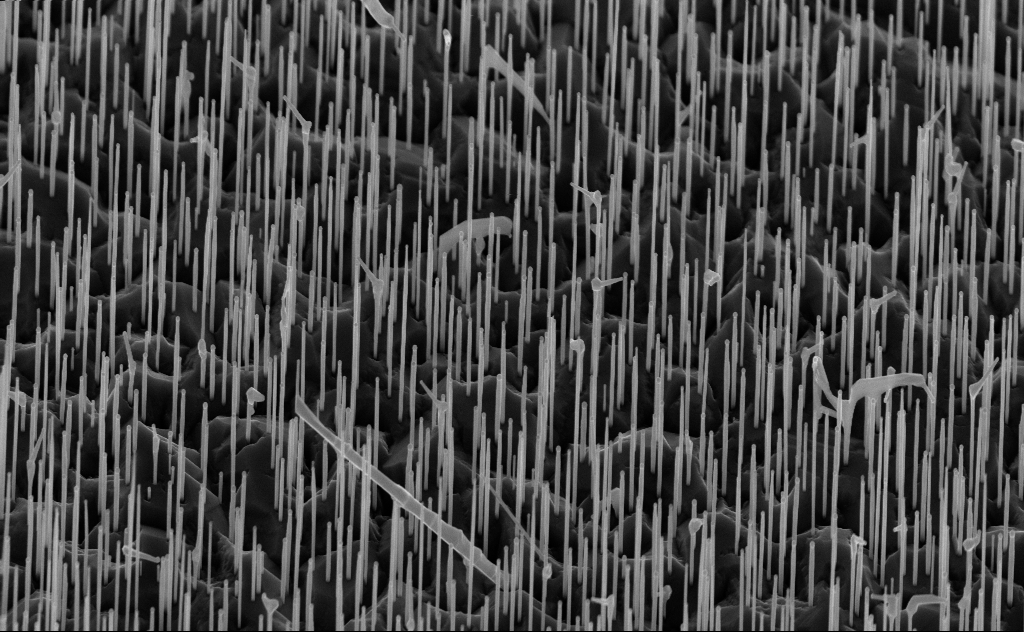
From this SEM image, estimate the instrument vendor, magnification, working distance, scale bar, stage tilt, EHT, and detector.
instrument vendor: Zeiss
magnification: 20 K X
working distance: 6 mm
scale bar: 1000 nm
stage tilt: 45°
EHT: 10 kV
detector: InLens